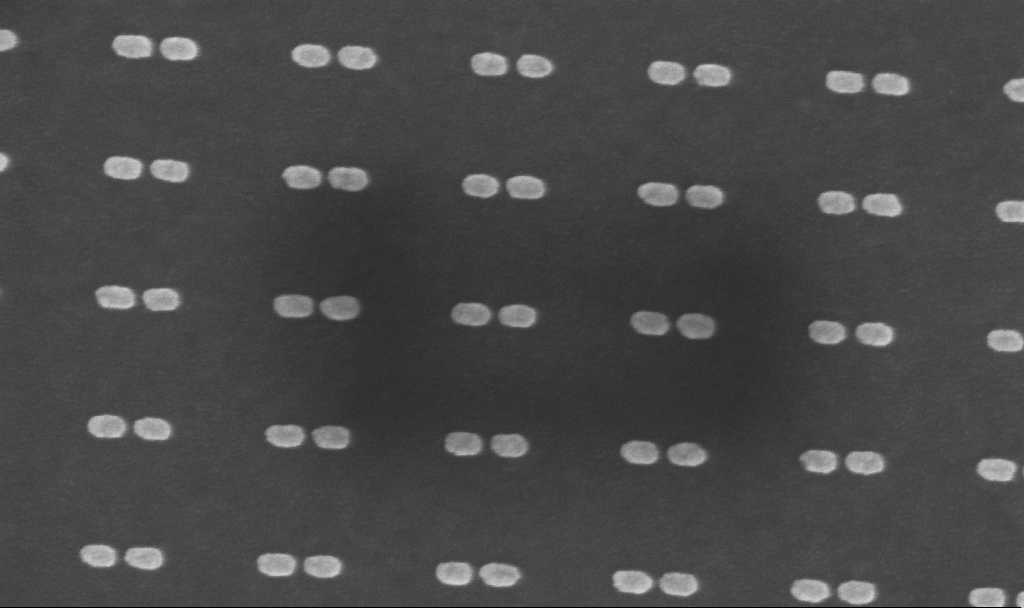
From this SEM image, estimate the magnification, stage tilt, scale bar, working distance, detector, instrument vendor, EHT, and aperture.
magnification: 161.58 K X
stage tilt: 0°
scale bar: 200 nm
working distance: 6 mm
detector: InLens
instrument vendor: Zeiss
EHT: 5 kV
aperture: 30 µm